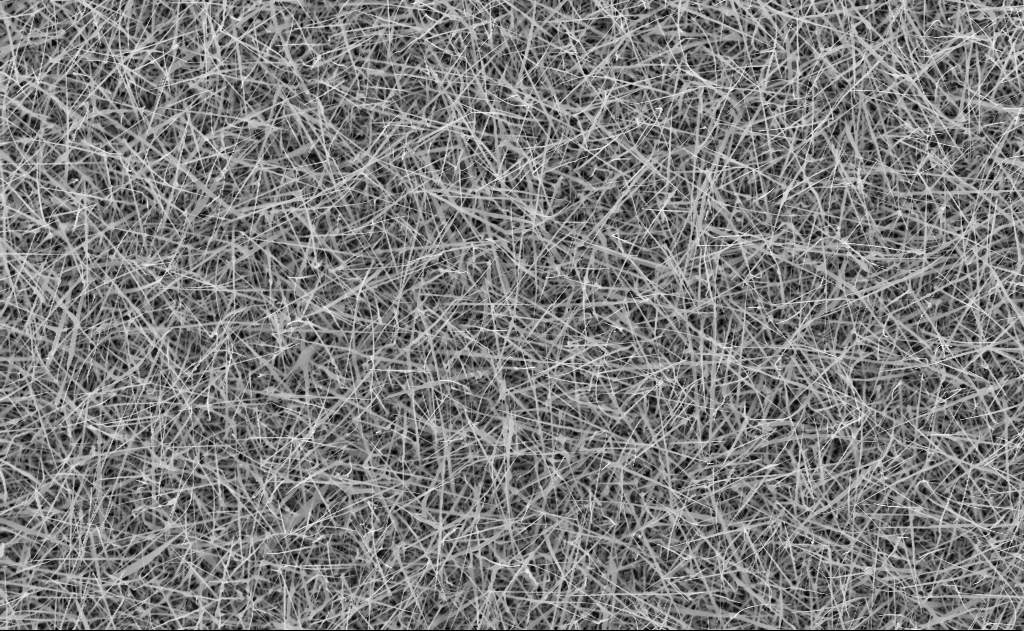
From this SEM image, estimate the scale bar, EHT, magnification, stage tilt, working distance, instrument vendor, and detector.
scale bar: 2000 nm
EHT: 10 kV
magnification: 10 K X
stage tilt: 0°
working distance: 10 mm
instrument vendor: Zeiss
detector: InLens